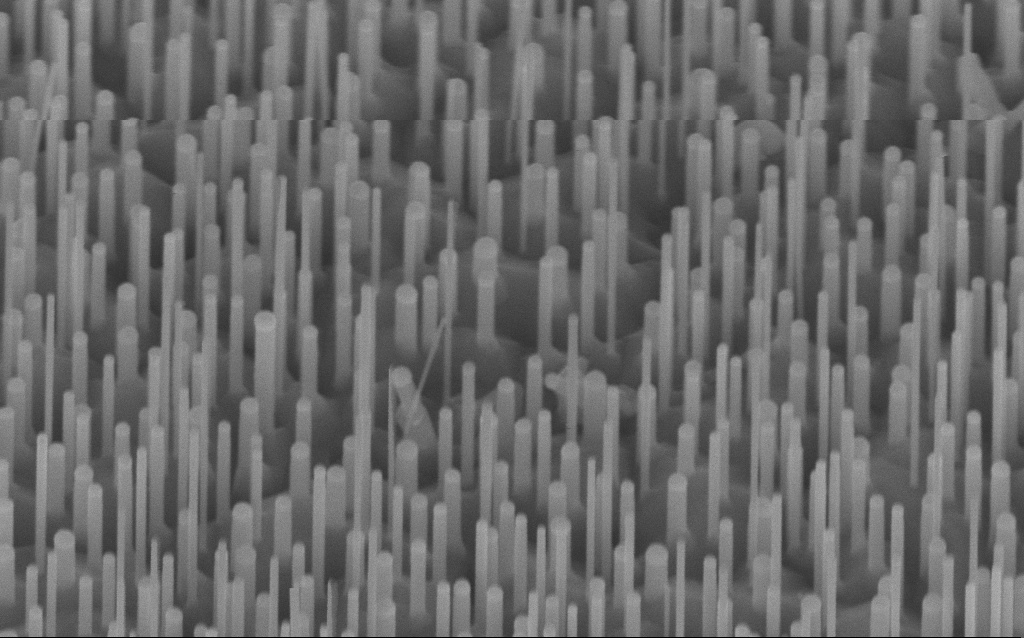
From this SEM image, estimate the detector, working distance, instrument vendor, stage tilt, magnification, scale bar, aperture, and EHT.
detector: InLens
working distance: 8 mm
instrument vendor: Zeiss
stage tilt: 45°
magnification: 80 K X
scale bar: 200 nm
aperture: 30 µm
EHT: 10 kV